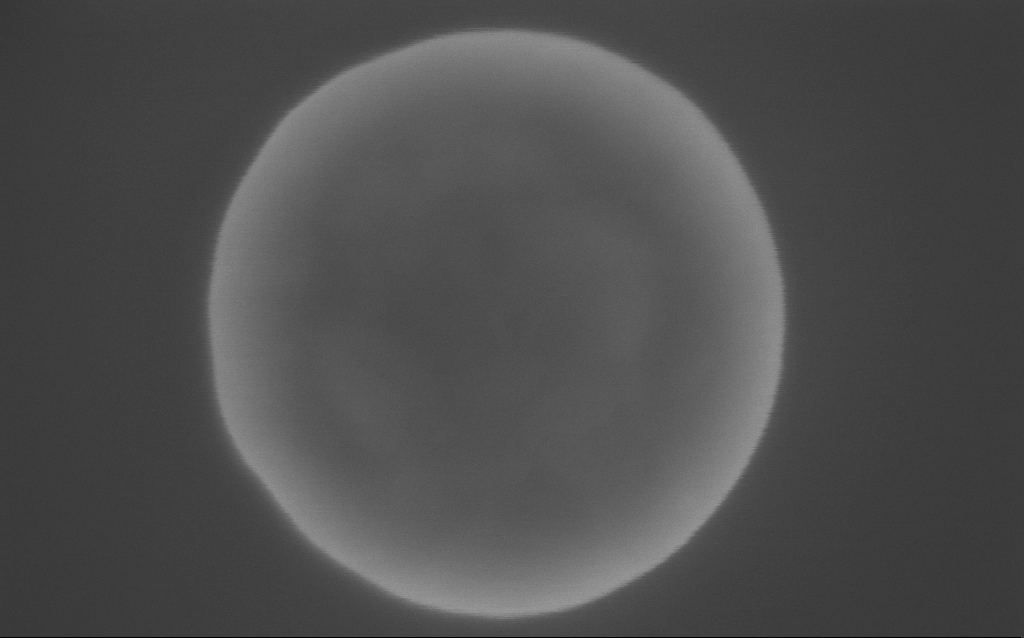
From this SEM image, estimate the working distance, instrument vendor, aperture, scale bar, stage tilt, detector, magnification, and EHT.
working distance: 2 mm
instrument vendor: Zeiss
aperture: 30 µm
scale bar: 200 nm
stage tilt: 0°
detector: InLens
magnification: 295.48 K X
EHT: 10 kV